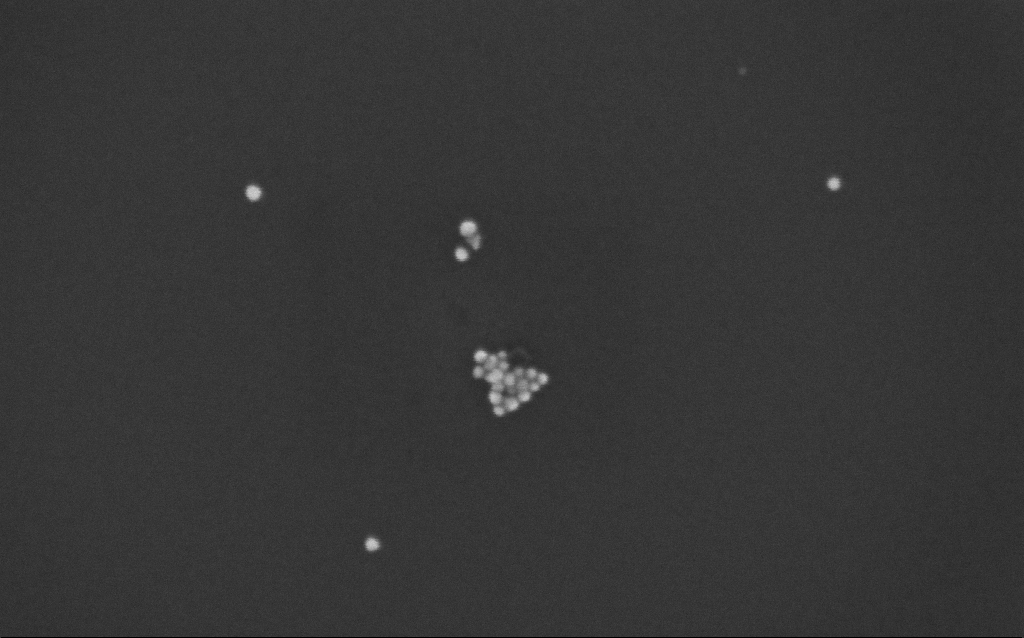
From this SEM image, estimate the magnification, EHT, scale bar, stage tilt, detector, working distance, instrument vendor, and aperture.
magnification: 312.14 K X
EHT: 10 kV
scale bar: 100 nm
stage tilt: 0°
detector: InLens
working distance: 7 mm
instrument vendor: Zeiss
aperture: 30 µm